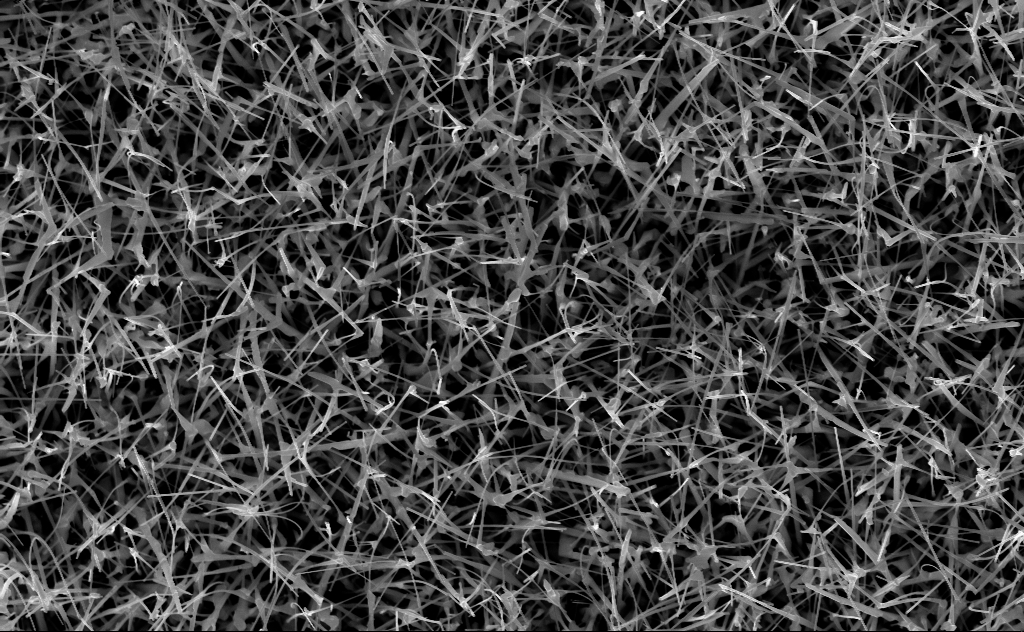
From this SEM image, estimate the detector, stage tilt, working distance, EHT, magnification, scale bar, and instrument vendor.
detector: InLens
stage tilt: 0°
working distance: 6 mm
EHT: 10 kV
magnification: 20 K X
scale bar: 2000 nm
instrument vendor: Zeiss